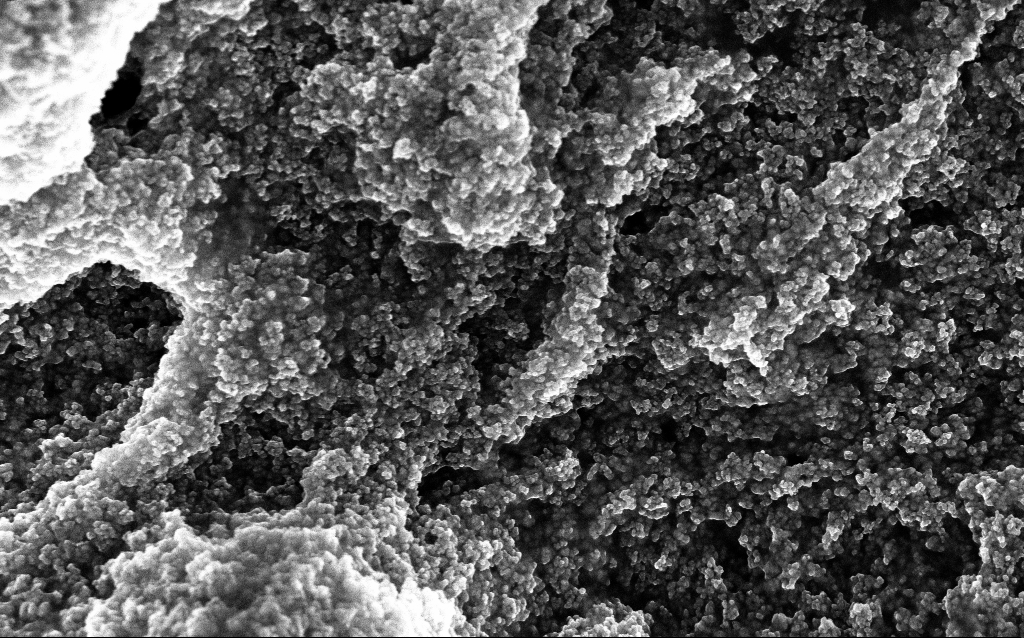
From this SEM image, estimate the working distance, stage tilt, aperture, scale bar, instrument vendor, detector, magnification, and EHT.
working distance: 2.6 mm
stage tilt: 0°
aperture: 30 µm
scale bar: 1000 nm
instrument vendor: Zeiss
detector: InLens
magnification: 65.04 K X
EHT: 10 kV